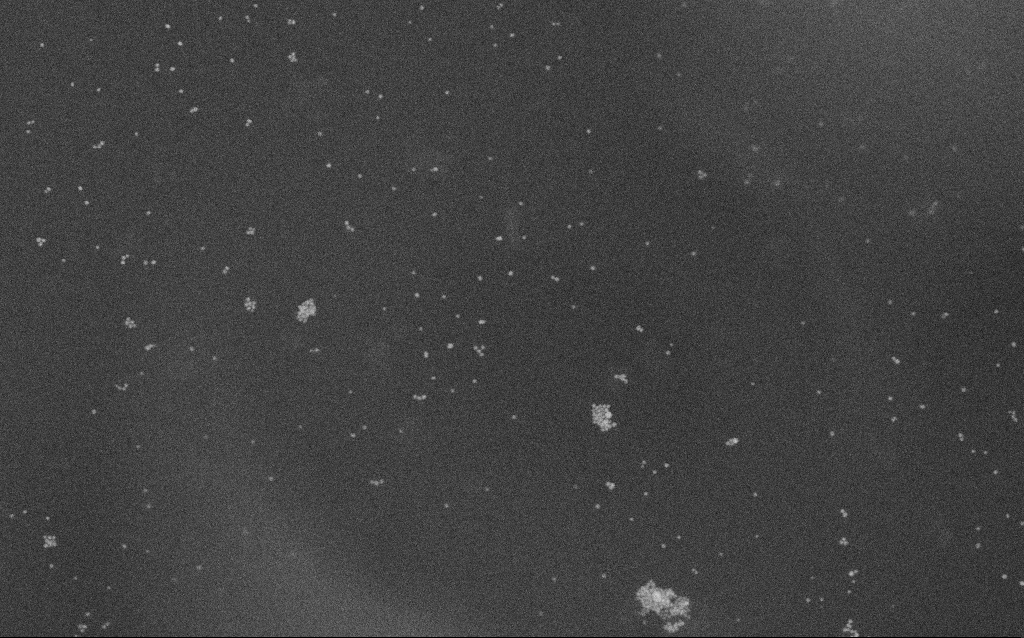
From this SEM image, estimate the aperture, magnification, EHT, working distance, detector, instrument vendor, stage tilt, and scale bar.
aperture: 30 µm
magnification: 100 K X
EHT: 10 kV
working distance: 2.1 mm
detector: SE2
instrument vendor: Zeiss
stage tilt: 0°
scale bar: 200 nm